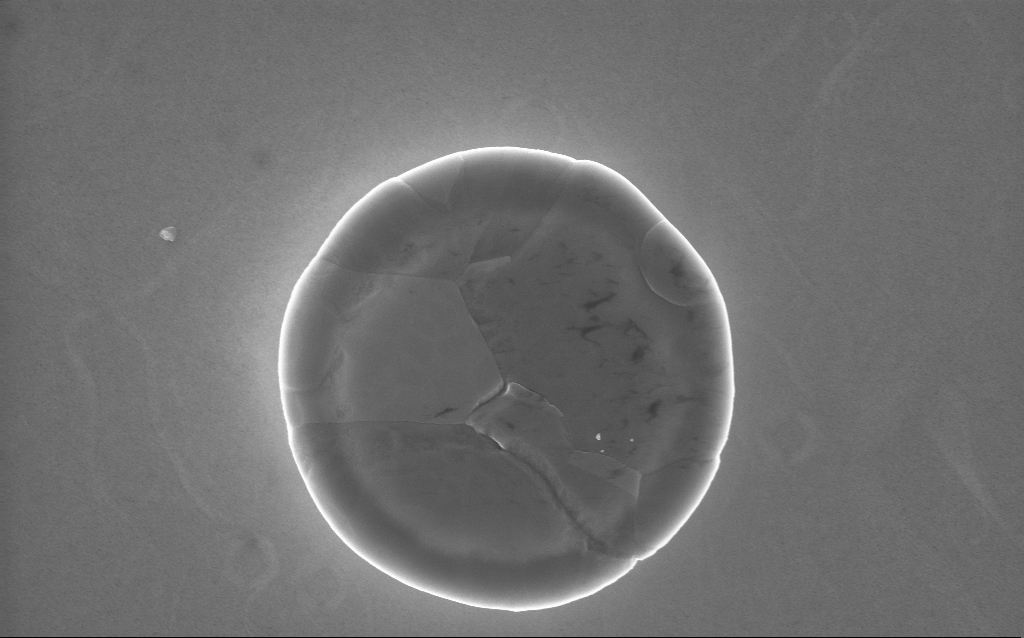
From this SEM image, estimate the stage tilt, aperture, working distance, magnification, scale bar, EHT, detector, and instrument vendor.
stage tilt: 0°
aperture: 30 µm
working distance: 2 mm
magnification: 33.71 K X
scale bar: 1000 nm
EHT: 10 kV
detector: InLens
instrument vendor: Zeiss